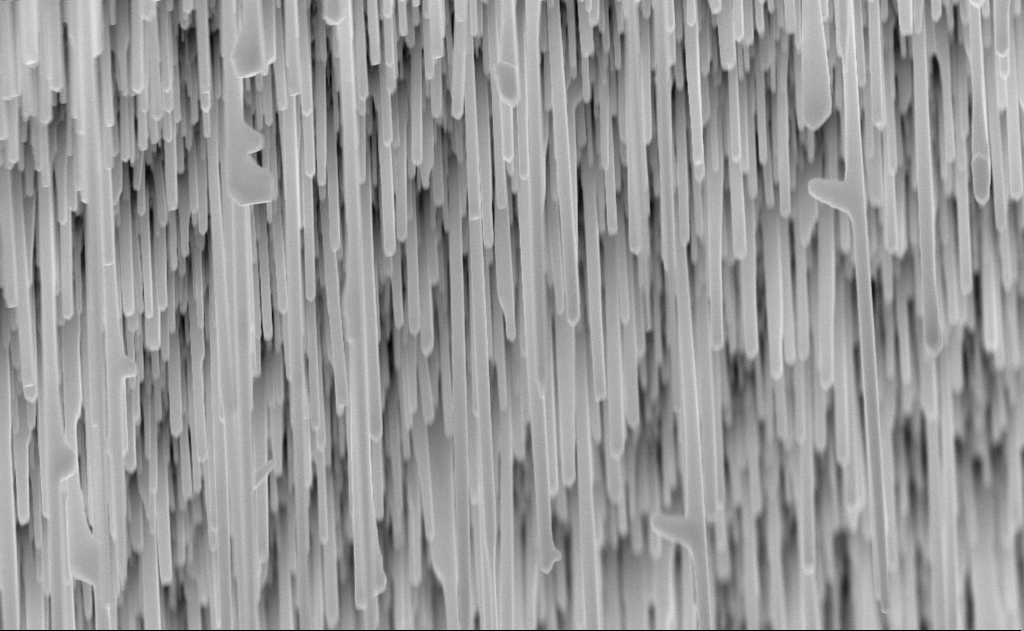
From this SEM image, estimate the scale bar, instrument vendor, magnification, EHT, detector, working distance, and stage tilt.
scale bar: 1000 nm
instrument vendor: Zeiss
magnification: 40 K X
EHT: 10 kV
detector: InLens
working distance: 6 mm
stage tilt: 0°